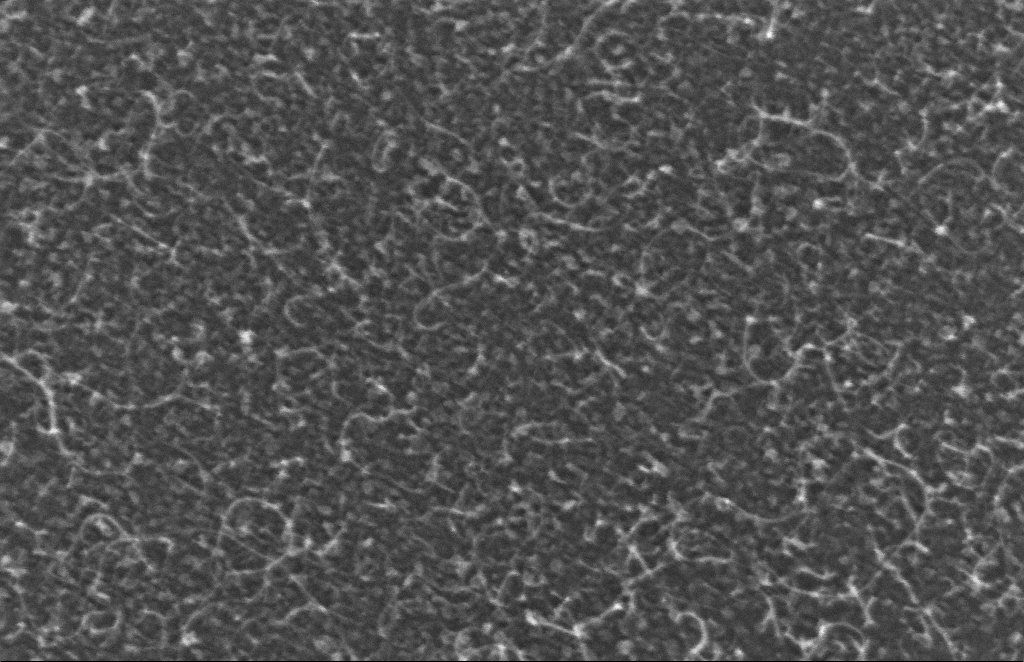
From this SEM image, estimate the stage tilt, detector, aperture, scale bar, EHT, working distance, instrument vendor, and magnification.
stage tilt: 0°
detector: InLens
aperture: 30 µm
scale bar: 100 nm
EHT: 5 kV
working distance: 6 mm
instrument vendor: Zeiss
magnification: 329.7 K X